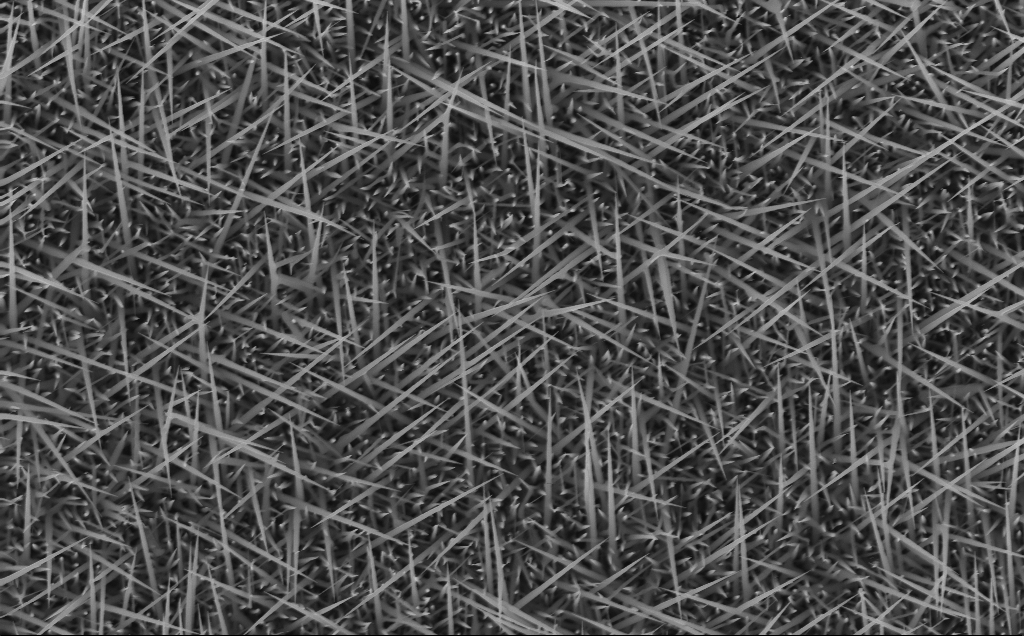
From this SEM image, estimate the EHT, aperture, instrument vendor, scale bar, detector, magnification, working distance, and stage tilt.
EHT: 10 kV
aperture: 30 µm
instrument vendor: Zeiss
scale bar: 1000 nm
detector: InLens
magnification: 40 K X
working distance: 6 mm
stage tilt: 0°